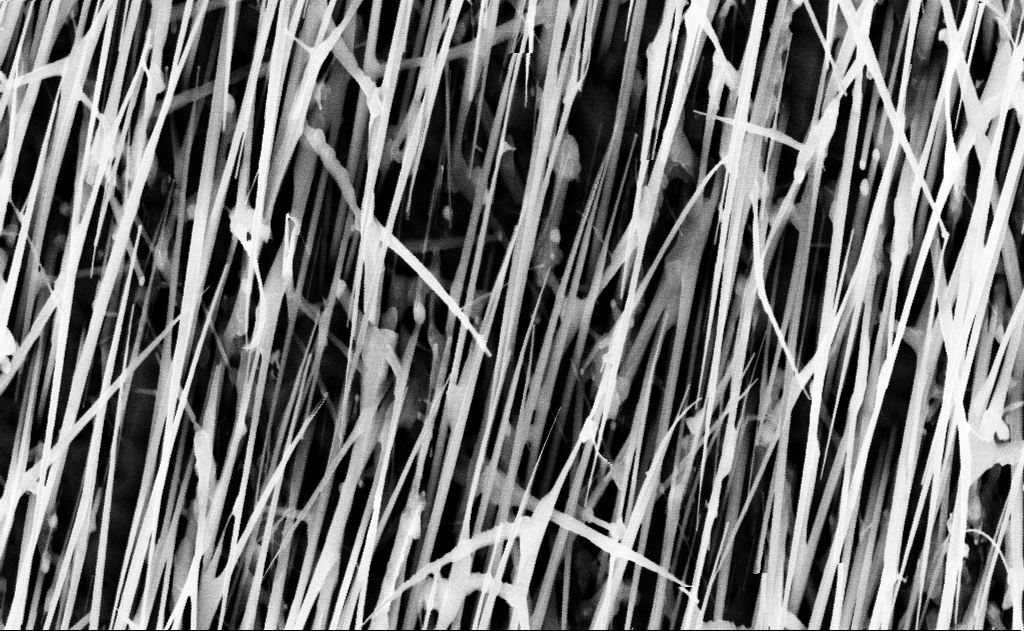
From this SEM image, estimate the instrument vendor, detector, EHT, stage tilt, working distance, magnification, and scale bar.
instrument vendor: Zeiss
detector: InLens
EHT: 10 kV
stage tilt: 0°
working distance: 16 mm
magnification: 40 K X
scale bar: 1000 nm